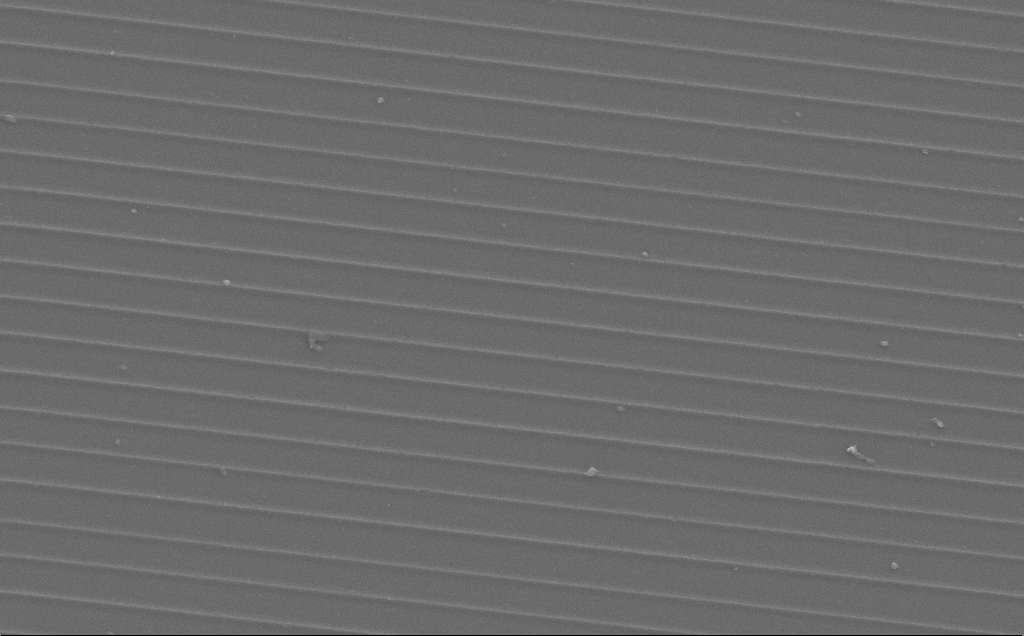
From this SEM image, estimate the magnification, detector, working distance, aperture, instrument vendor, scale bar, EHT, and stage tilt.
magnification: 2.03 K X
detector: SE2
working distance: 11 mm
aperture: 30 µm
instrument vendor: Zeiss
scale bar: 20000 nm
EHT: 10 kV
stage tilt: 0°